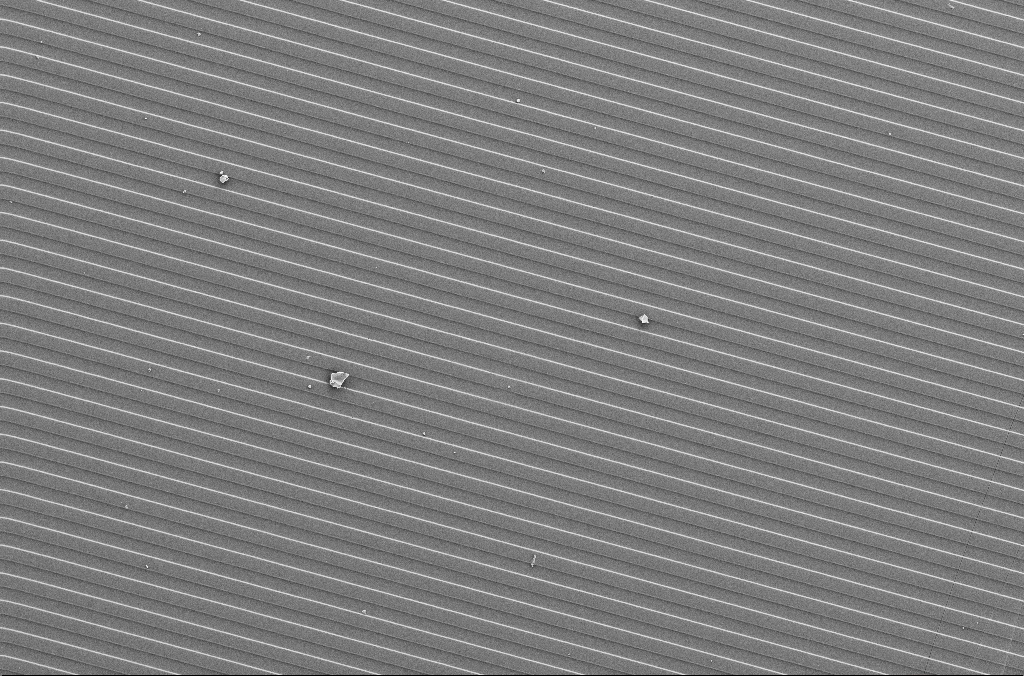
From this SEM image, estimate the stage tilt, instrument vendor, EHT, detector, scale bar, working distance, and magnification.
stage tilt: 0°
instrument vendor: Zeiss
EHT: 5 kV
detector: SE2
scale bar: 100000 nm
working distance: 4.1 mm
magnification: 0.5 K X